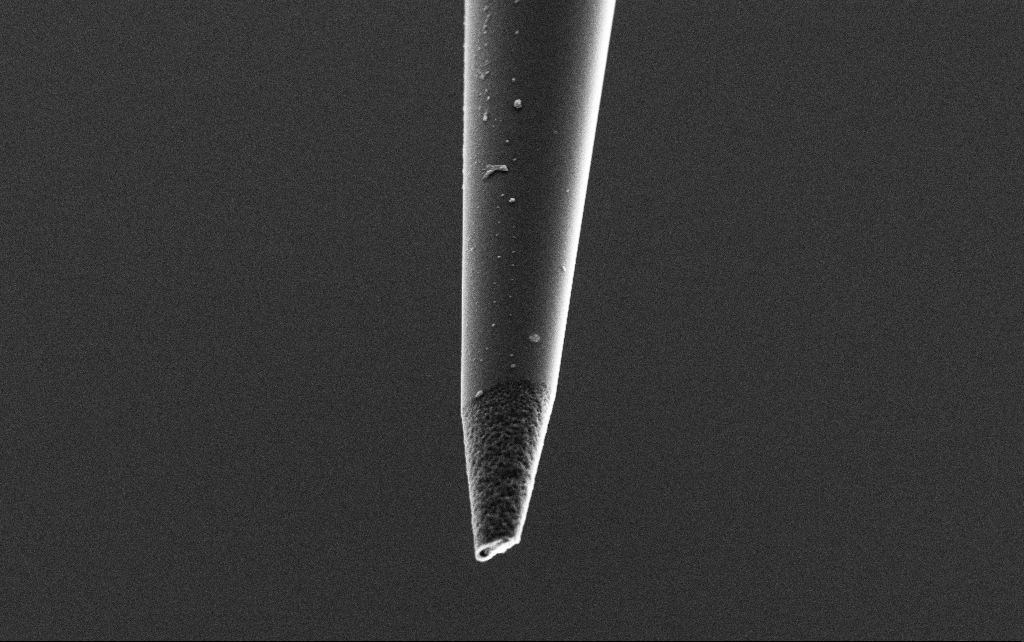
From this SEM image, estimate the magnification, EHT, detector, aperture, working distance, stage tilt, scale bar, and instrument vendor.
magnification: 15 K X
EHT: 3 kV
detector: SE2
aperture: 30 µm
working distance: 7.7 mm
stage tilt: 45°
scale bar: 2000 nm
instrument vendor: Zeiss